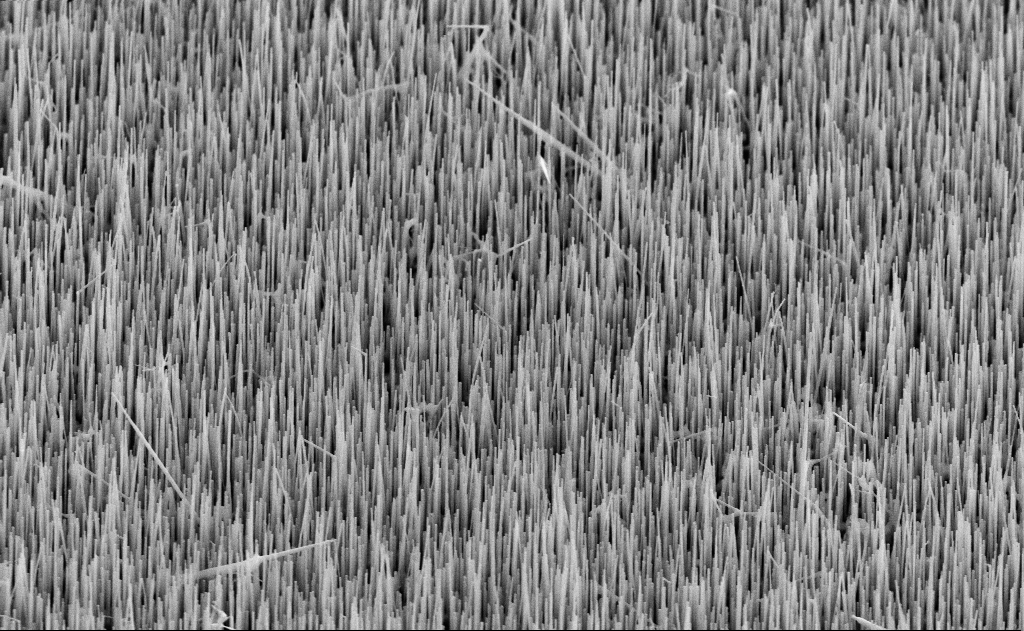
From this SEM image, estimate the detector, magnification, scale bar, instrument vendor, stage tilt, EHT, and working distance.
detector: SE2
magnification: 20 K X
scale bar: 1000 nm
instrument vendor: Zeiss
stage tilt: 45°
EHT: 10 kV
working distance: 11 mm